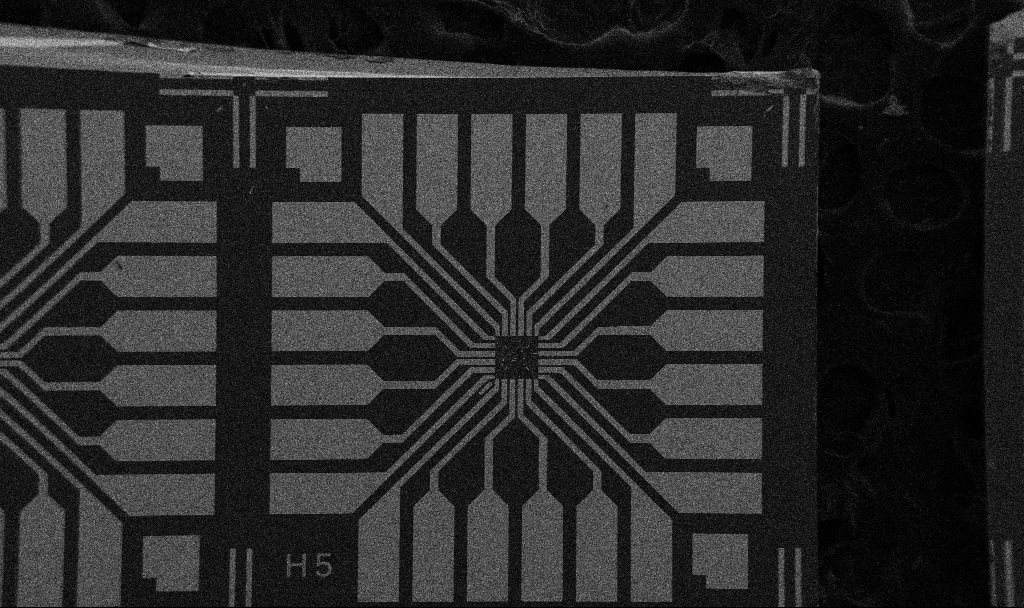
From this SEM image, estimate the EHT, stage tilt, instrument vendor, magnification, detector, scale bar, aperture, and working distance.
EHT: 5 kV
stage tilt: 0°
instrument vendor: Zeiss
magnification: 0.1 K X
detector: SE2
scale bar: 200000 nm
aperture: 30 µm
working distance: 10.7 mm